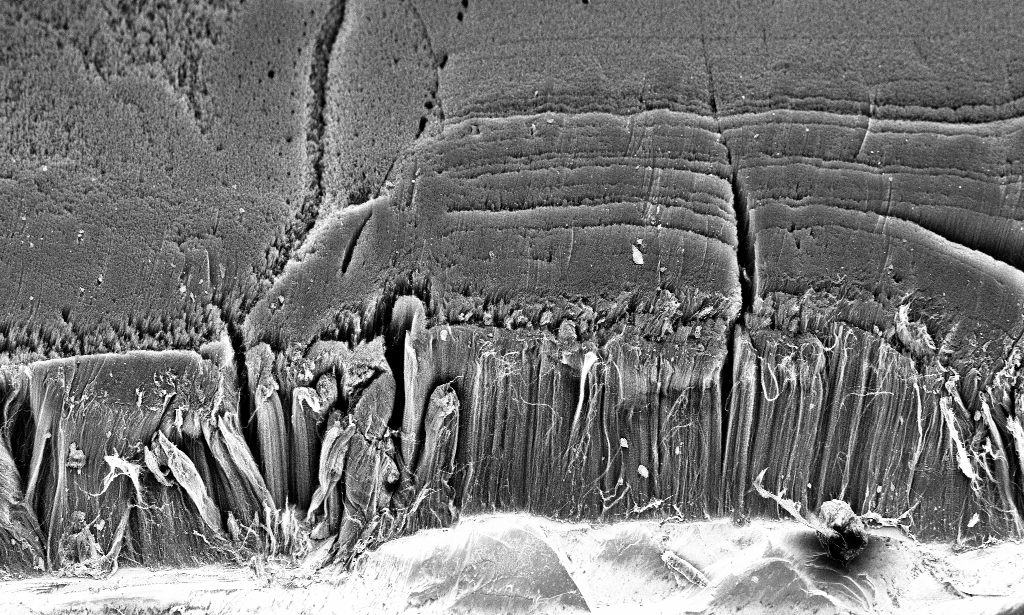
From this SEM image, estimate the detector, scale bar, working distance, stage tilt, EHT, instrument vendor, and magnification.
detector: InLens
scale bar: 100000 nm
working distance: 3.3 mm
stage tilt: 45°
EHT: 3 kV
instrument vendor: Zeiss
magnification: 0.6 K X